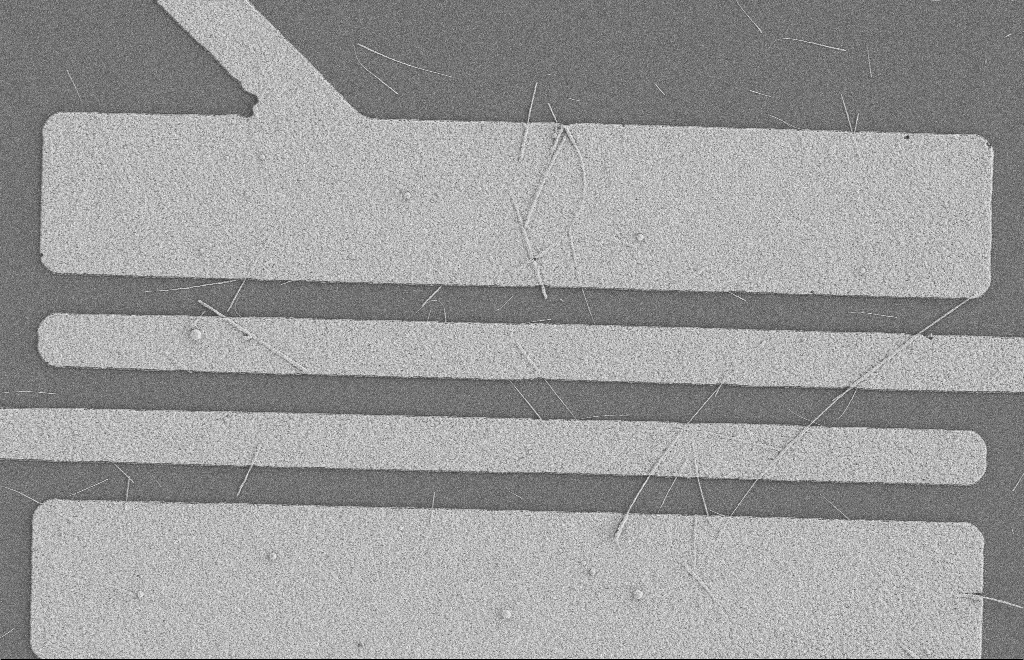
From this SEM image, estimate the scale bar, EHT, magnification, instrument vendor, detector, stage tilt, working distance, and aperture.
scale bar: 2000 nm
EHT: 2 kV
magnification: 5.71 K X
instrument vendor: Zeiss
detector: SE2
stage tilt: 0°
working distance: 8 mm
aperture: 20 µm